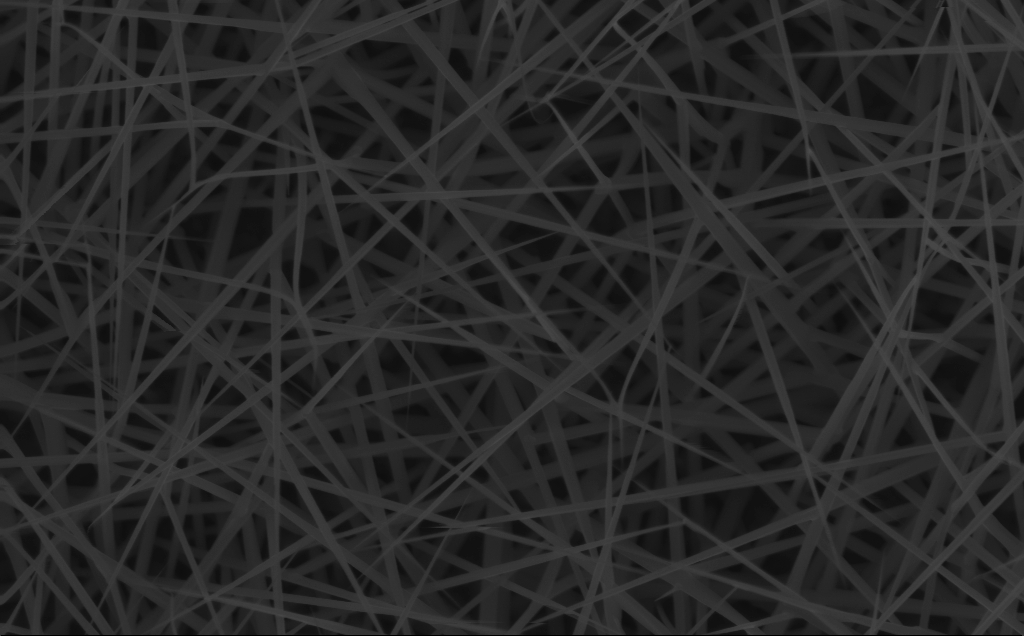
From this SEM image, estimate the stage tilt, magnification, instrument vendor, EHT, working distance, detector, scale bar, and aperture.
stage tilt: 0°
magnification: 40 K X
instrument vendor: Zeiss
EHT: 10 kV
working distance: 4 mm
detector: InLens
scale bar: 1000 nm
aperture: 30 µm